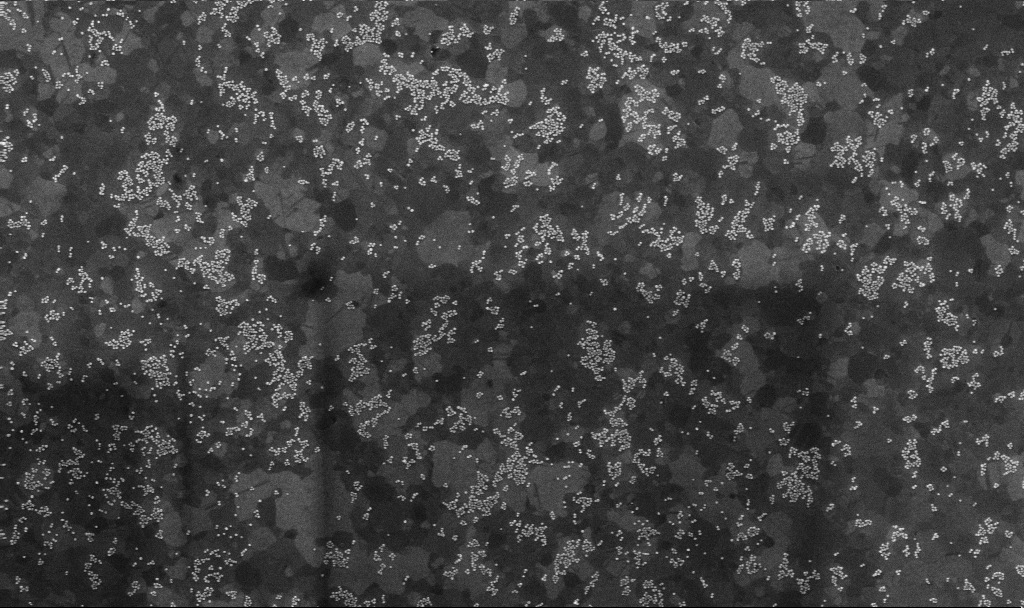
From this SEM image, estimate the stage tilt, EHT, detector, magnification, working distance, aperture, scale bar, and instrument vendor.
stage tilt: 0°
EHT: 10 kV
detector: InLens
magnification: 50 K X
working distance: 3.8 mm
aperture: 30 µm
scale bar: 1000 nm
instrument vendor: Zeiss